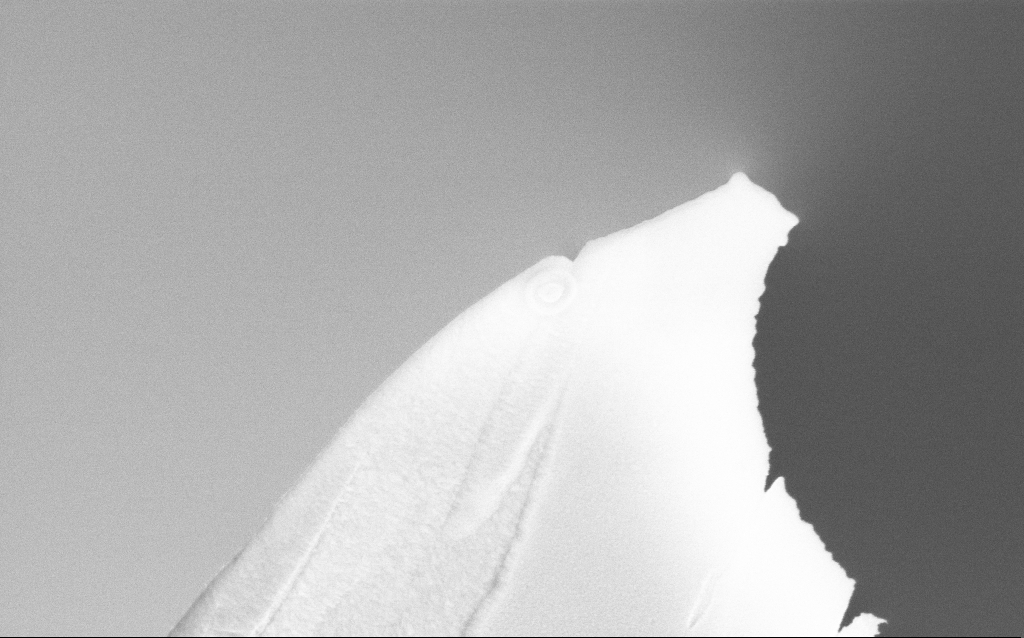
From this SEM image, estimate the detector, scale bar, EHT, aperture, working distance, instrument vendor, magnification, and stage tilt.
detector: InLens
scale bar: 100 nm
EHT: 5 kV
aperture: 30 µm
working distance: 2.9 mm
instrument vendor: Zeiss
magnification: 159.69 K X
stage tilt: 0°